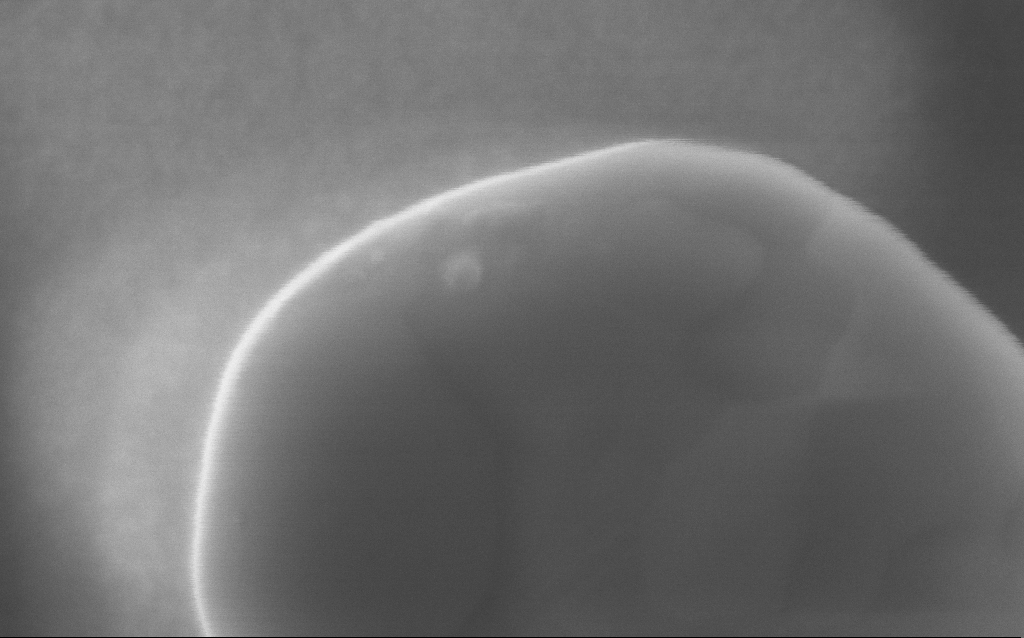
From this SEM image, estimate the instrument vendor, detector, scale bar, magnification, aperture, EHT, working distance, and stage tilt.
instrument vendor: Zeiss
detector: InLens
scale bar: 100 nm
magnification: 350 K X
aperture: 30 µm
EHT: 5 kV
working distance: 4 mm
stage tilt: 0°